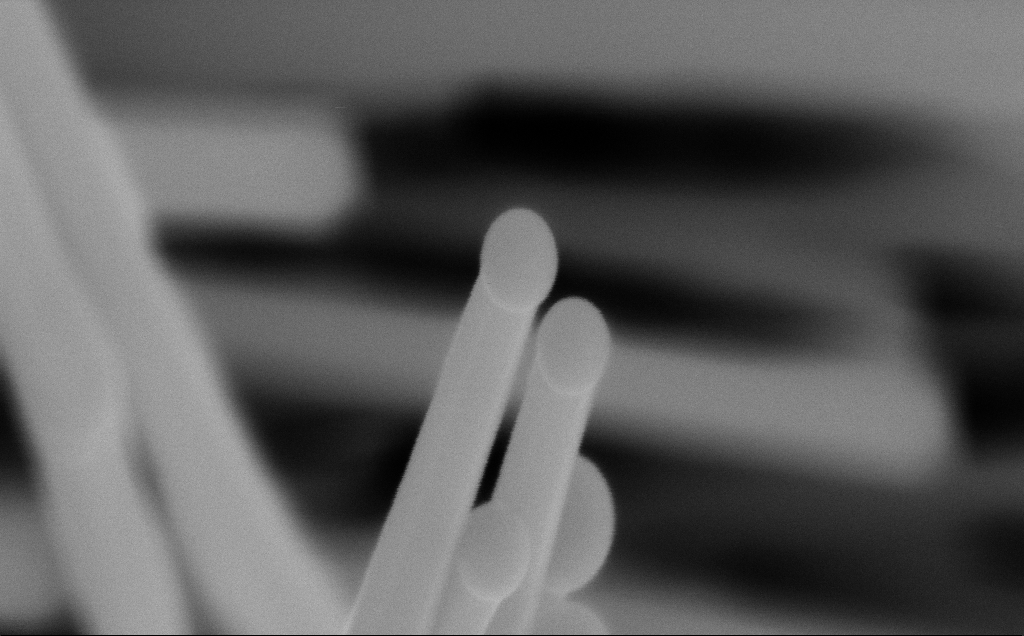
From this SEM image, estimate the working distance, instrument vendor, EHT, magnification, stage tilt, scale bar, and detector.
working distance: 6 mm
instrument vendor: Zeiss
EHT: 10 kV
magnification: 339.78 K X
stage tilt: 0°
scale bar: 200 nm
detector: InLens